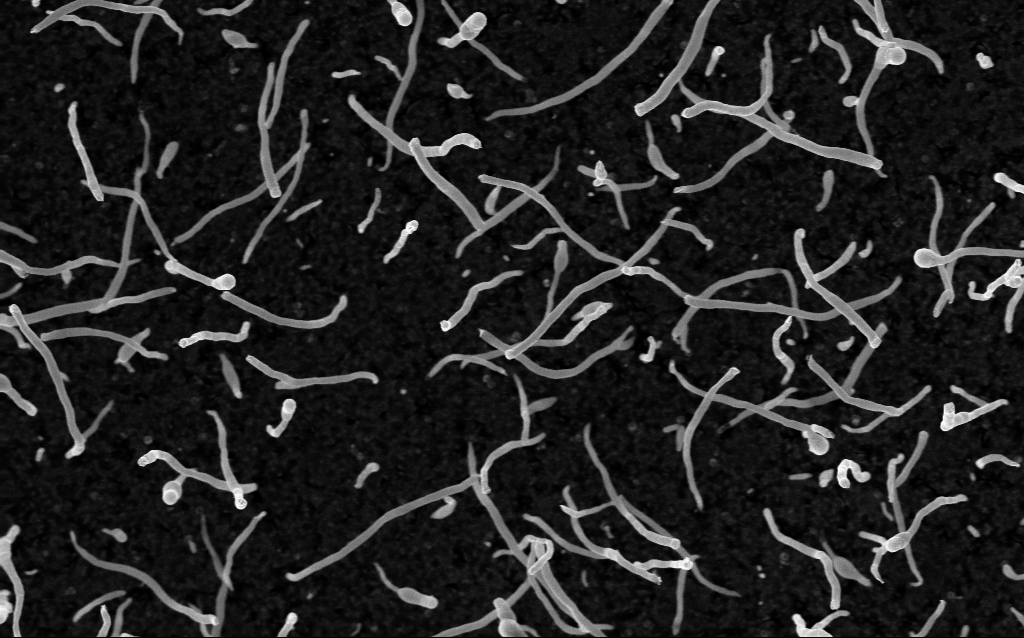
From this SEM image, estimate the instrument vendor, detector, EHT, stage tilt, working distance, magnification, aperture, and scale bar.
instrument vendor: Zeiss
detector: InLens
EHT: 5 kV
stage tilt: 0°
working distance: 1.8 mm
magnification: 50 K X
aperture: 30 µm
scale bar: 1000 nm